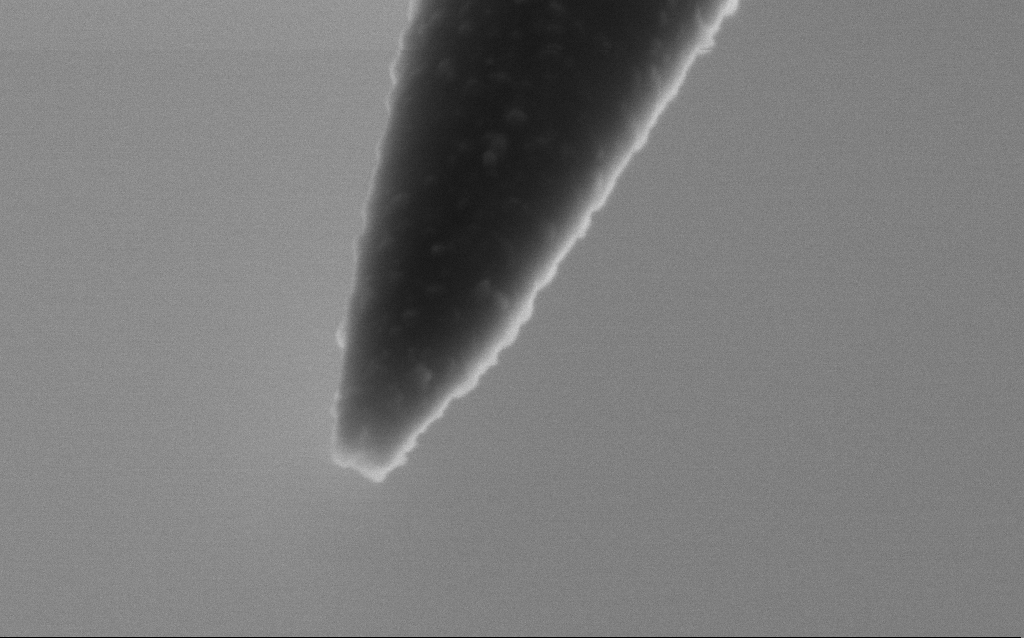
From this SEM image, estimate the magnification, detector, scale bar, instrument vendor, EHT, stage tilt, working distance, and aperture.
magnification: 250 K X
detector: SE2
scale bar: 200 nm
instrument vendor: Zeiss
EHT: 2.5 kV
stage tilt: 45°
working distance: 5 mm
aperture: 30 µm